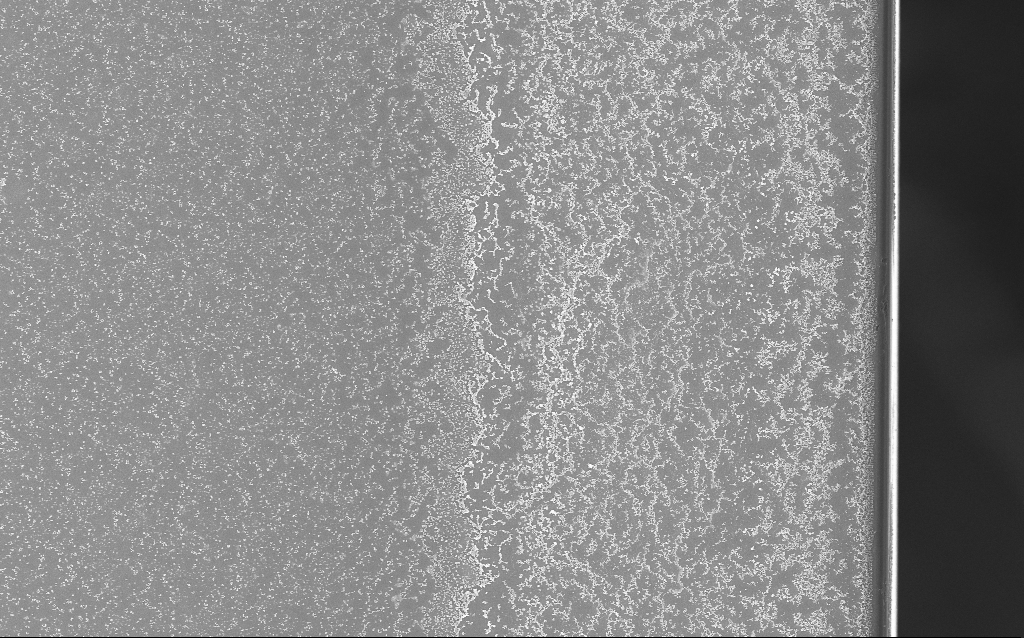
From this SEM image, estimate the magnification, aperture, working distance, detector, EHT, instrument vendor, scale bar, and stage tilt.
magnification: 1 K X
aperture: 30 µm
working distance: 1.7 mm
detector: InLens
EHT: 20 kV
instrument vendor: Zeiss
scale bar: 20000 nm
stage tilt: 0°